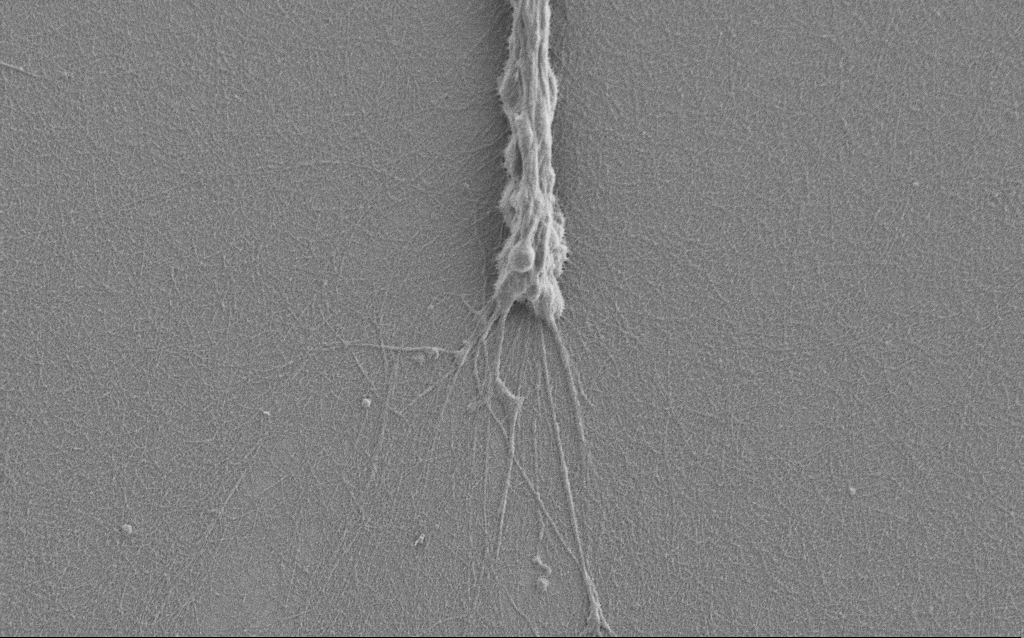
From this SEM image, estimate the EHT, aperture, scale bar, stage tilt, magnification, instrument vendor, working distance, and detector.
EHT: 1 kV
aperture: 30 µm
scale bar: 2000 nm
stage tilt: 0°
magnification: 7.5 K X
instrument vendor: Zeiss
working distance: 6 mm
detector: SE2